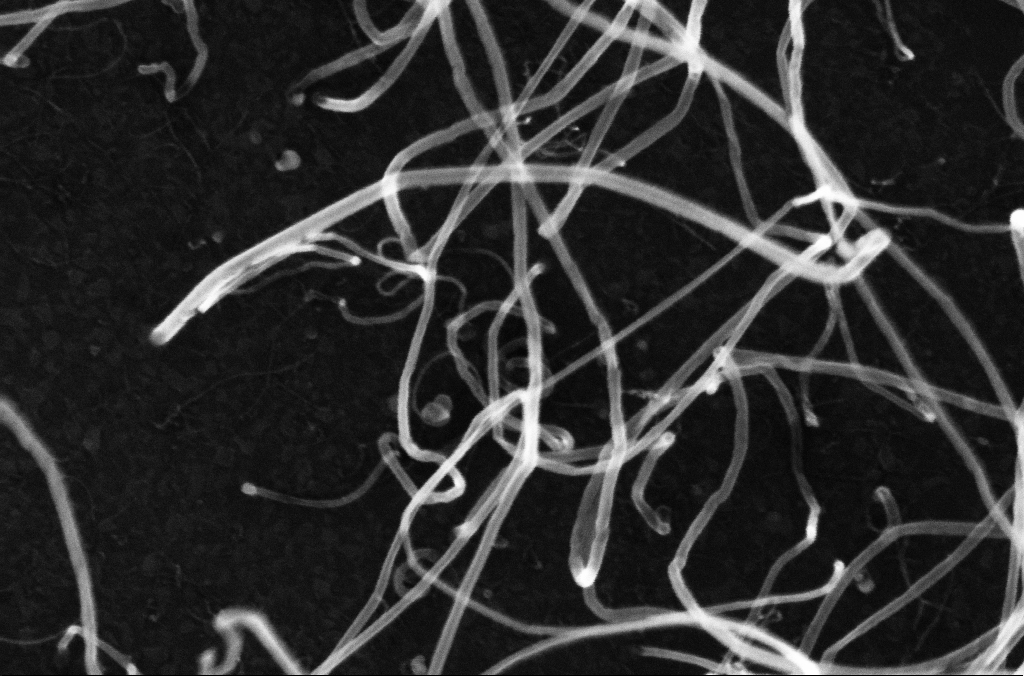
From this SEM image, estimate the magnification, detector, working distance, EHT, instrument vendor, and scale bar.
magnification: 200 K X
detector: InLens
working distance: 3.3 mm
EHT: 10 kV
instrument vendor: Zeiss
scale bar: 200 nm